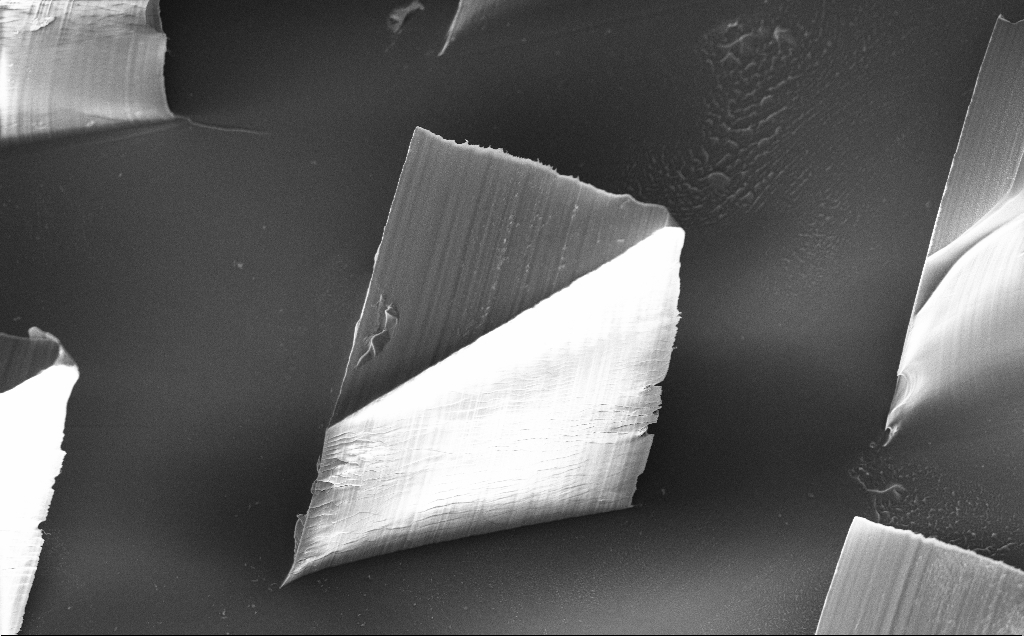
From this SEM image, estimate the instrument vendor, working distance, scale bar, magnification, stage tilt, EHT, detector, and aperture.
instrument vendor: Zeiss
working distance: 8 mm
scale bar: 100000 nm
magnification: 0.5 K X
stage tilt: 20°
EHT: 10 kV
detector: InLens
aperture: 30 µm